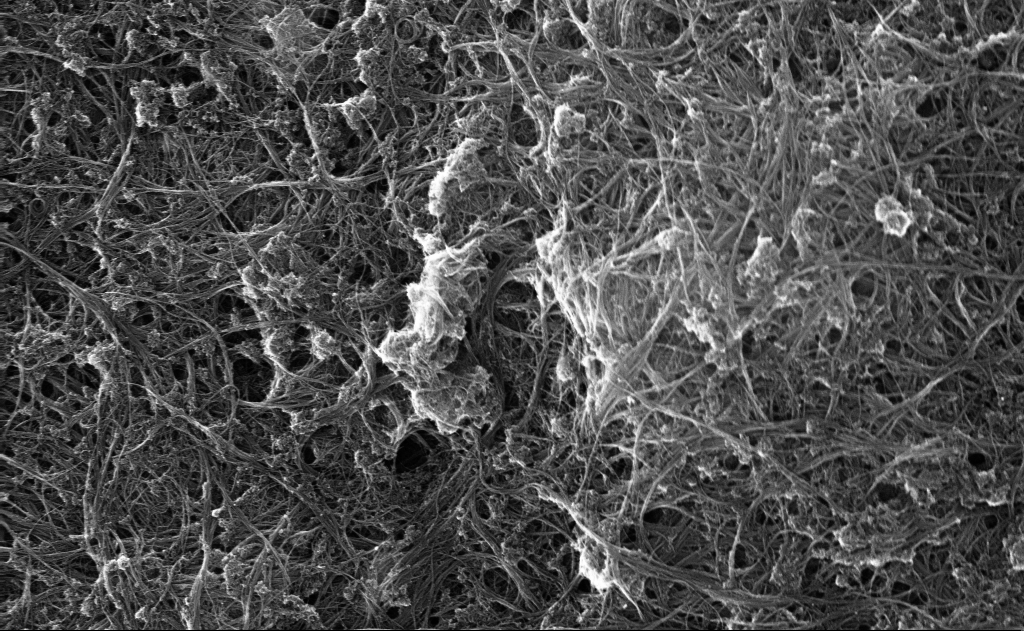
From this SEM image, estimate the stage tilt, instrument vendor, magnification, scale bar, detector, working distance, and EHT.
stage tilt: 0°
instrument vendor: Zeiss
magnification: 40.57 K X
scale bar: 1000 nm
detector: InLens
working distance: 6 mm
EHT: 10 kV